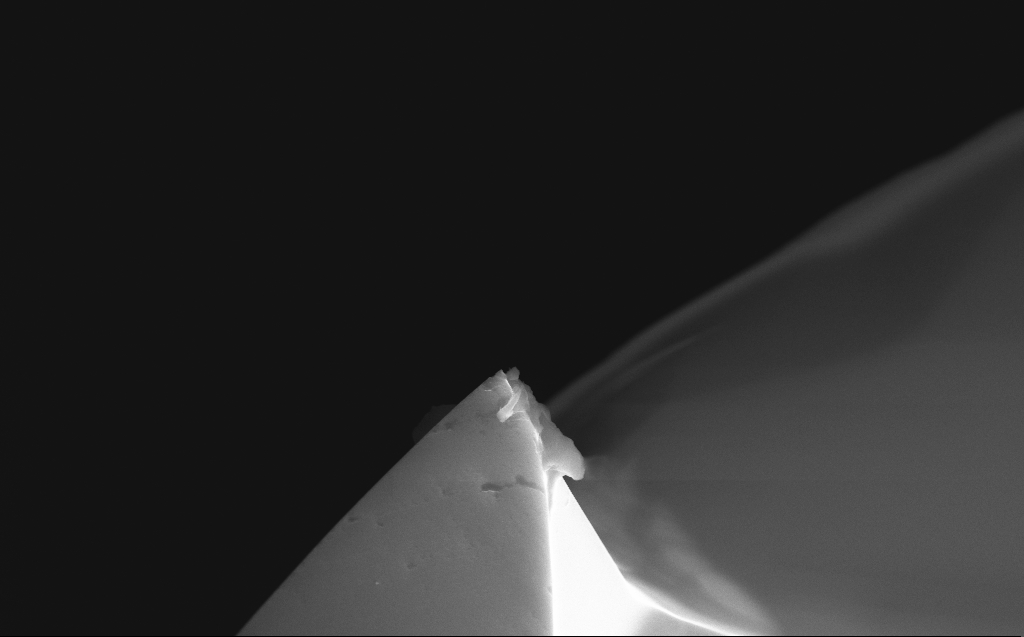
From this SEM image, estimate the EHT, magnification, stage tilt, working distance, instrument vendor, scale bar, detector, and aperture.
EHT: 10 kV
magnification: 15.18 K X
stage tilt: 35.7°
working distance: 6 mm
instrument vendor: Zeiss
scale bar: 1000 nm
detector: InLens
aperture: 30 µm